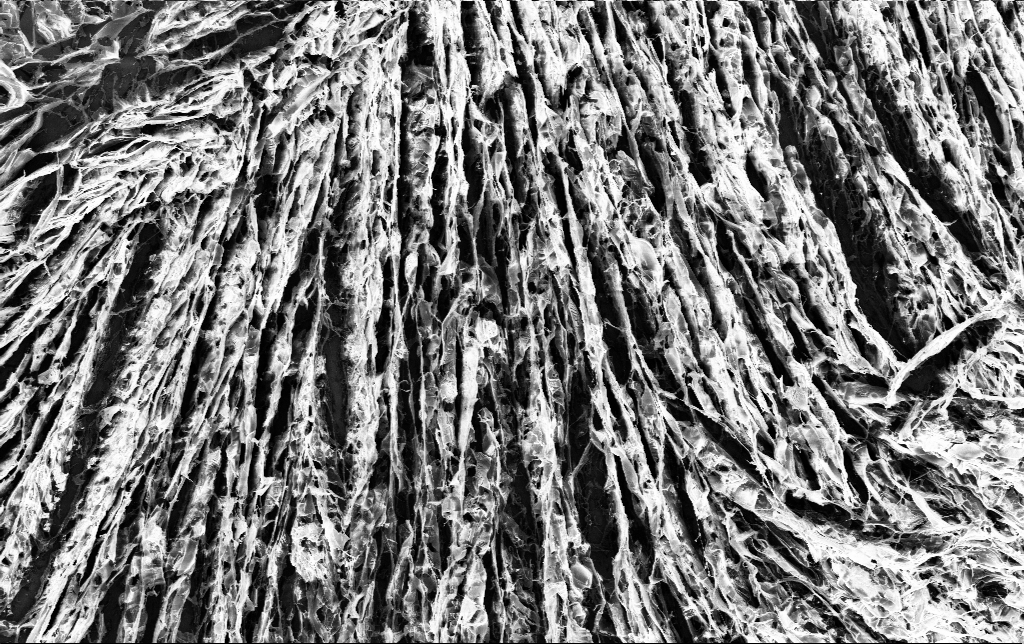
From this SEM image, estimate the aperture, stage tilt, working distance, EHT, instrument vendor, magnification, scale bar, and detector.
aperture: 30 µm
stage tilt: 0°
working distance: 3.4 mm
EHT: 3 kV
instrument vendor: Zeiss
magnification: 1.62 K X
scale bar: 20000 nm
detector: InLens